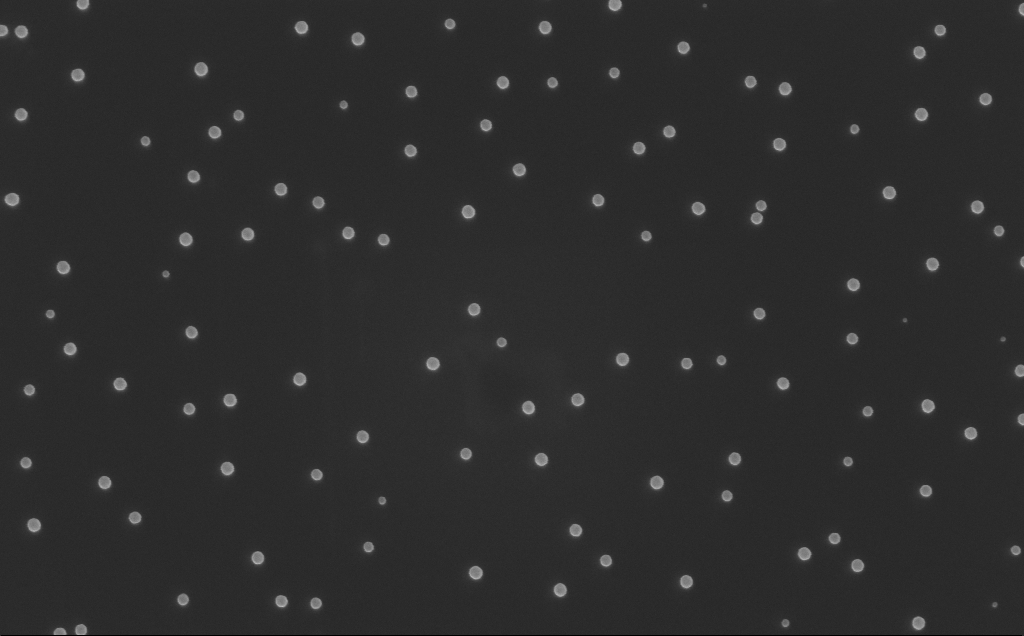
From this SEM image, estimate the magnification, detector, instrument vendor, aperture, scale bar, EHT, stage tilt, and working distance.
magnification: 80 K X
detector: InLens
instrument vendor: Zeiss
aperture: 30 µm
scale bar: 200 nm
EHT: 10 kV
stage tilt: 0°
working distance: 8 mm